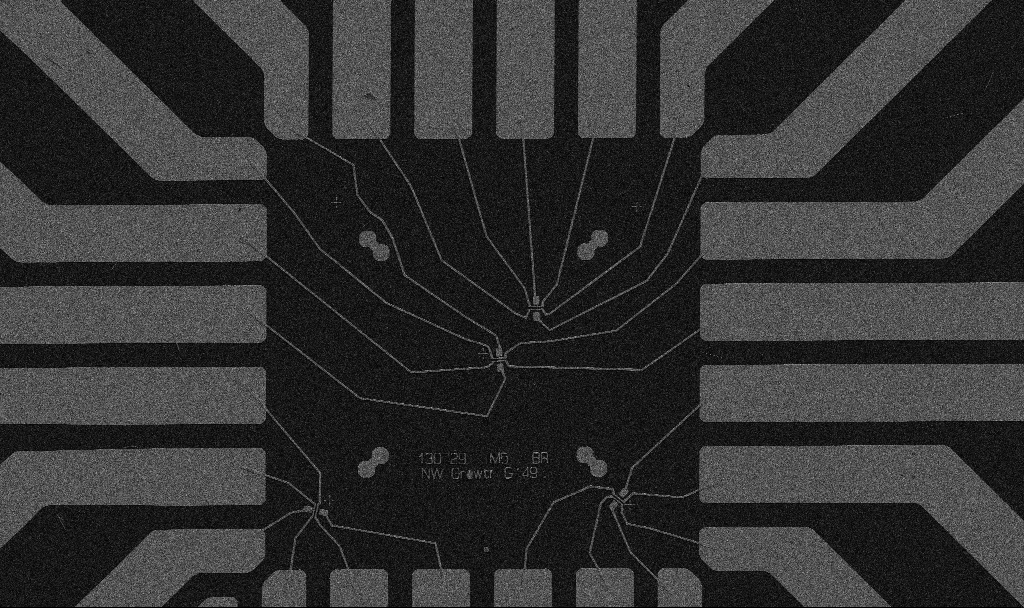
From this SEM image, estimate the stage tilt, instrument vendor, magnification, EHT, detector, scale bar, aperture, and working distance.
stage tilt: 0°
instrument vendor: Zeiss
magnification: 1 K X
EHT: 5 kV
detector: SE2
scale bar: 20000 nm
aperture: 30 µm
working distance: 10.7 mm